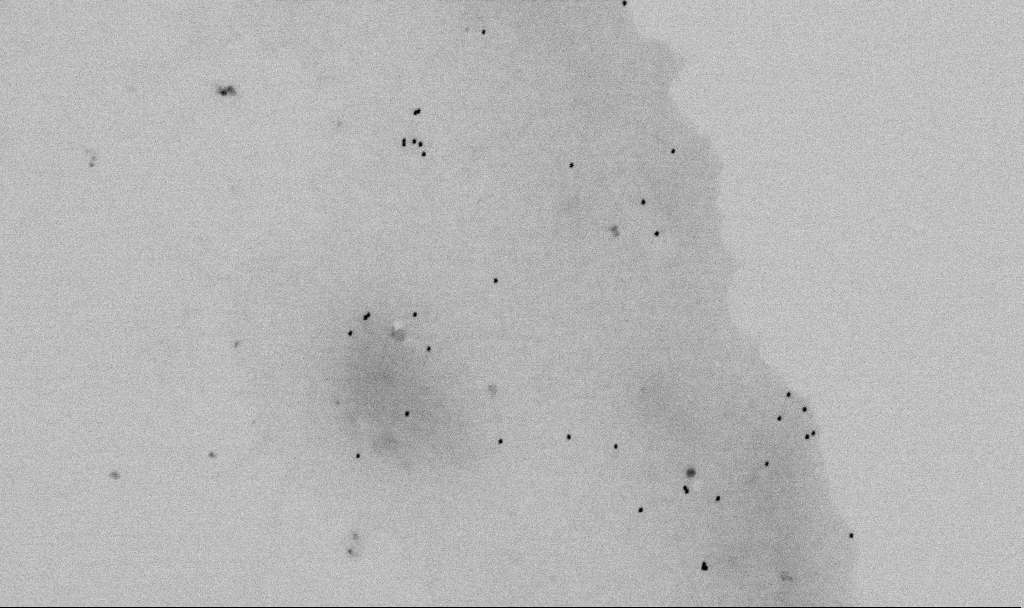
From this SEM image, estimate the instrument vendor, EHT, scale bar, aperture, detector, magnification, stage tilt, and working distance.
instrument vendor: Zeiss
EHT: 2 kV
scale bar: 1000 nm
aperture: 30 µm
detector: SE2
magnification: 60 K X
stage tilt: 0°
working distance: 5.5 mm